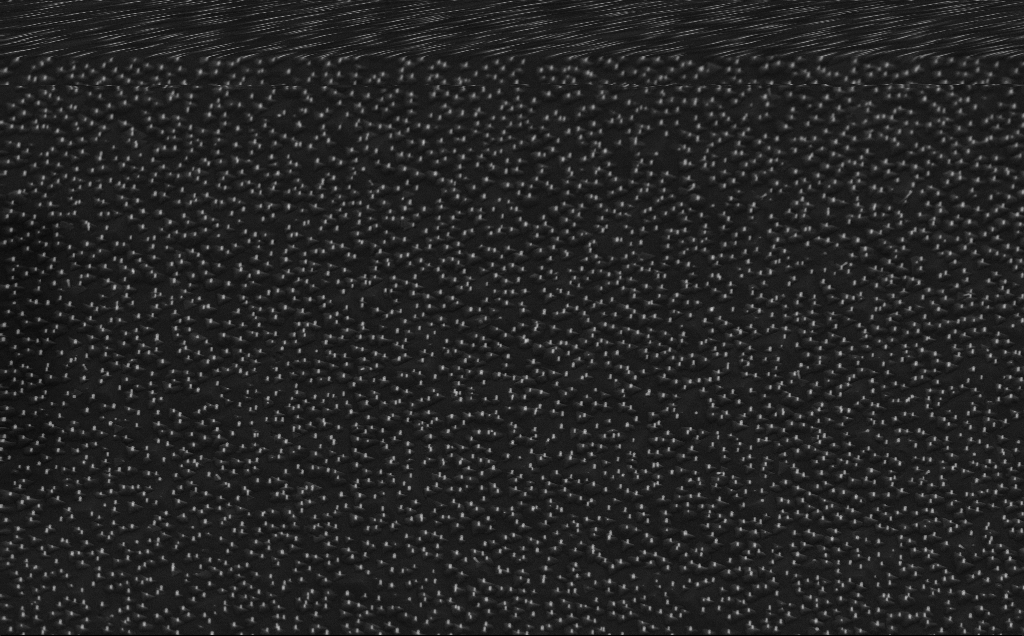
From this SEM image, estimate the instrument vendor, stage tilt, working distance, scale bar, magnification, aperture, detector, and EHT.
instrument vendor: Zeiss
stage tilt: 45°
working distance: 6 mm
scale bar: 1000 nm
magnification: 20 K X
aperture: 30 µm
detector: InLens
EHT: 10 kV